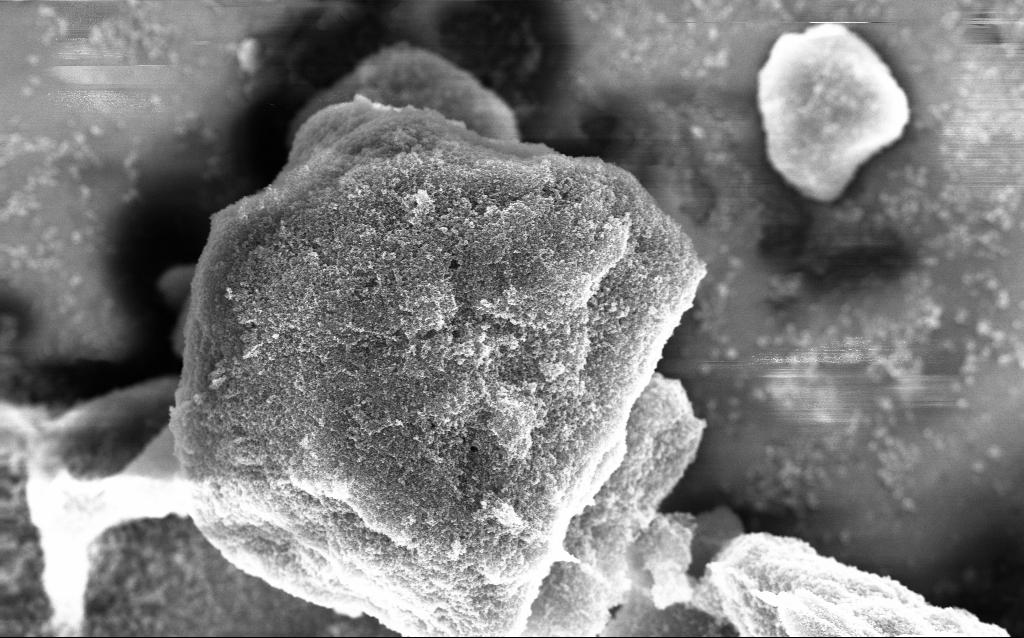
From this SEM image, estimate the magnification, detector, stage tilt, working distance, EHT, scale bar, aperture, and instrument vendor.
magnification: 20.87 K X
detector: InLens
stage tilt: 0°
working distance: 2.8 mm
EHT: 10 kV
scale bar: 1000 nm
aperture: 30 µm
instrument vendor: Zeiss